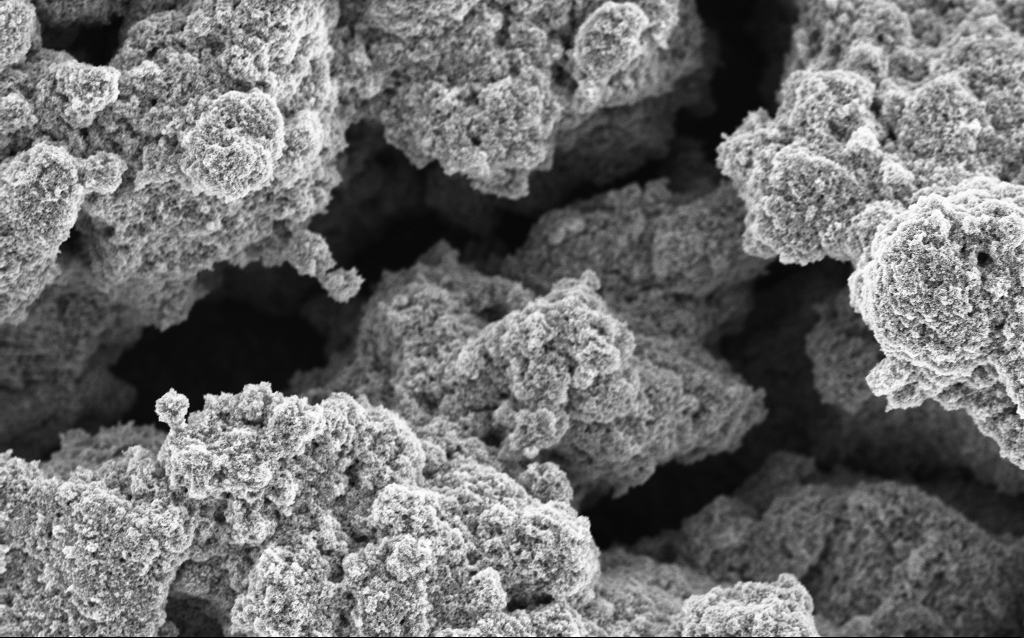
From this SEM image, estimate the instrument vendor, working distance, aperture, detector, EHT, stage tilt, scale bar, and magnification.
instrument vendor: Zeiss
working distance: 4.5 mm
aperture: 30 µm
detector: InLens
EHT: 5 kV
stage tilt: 0°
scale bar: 10000 nm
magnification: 6.4 K X